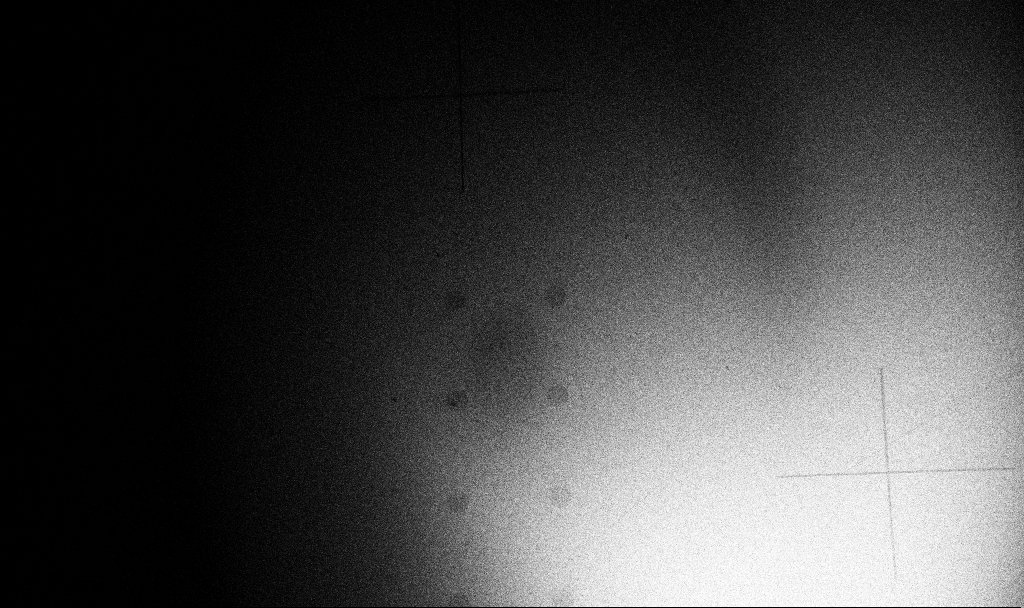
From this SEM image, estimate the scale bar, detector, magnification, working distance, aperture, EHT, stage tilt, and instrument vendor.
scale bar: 200000 nm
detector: InLens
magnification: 0.074 K X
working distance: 6.8 mm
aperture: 30 µm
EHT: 5 kV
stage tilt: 45°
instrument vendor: Zeiss